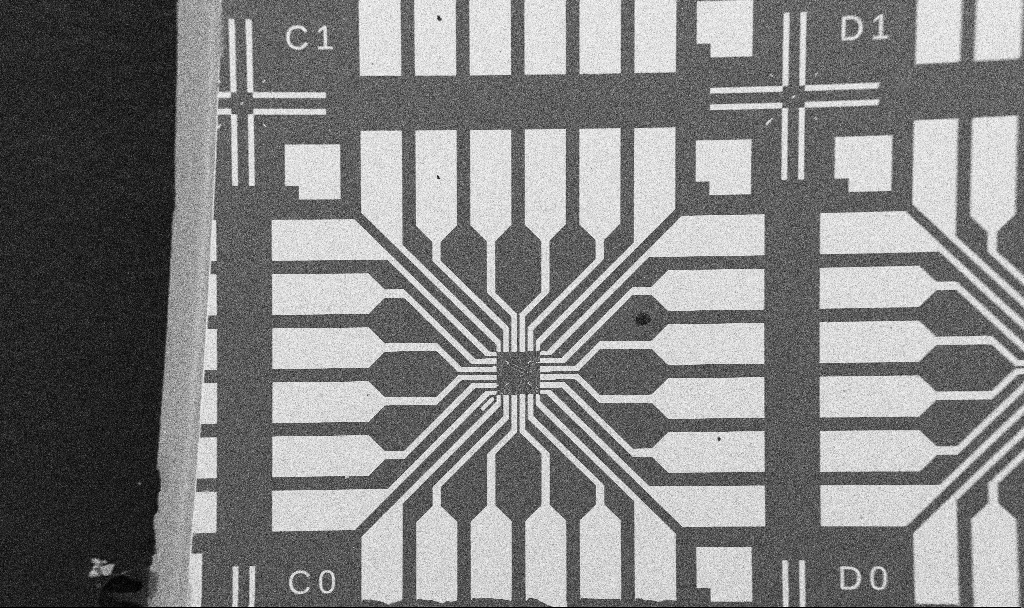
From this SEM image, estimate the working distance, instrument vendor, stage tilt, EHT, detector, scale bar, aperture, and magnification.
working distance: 10.7 mm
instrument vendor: Zeiss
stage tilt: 0°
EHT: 5 kV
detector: SE2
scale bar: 200000 nm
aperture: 30 µm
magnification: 0.1 K X